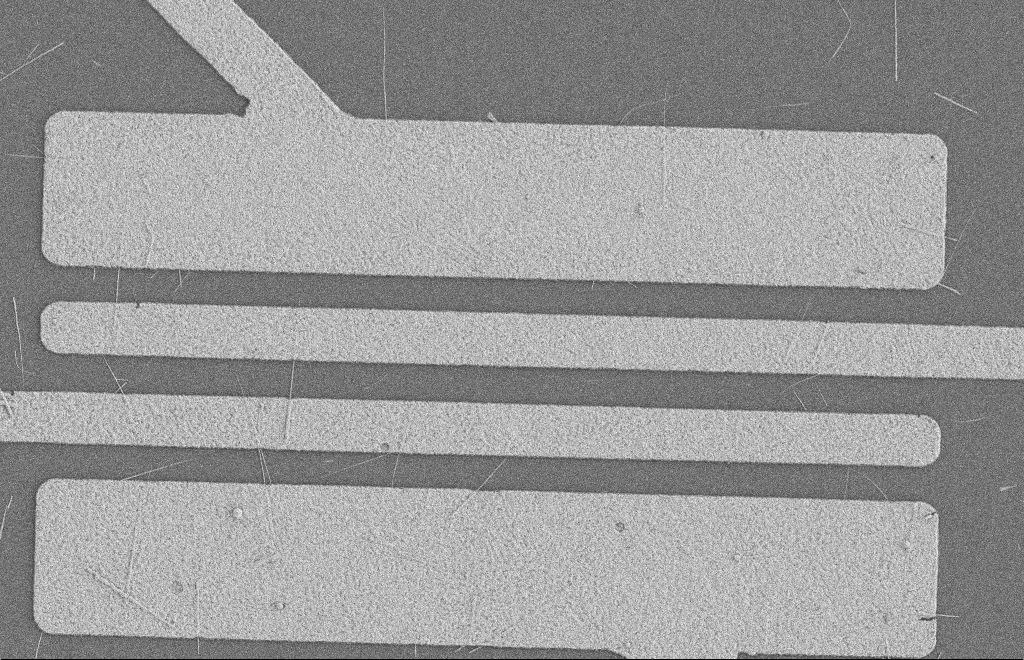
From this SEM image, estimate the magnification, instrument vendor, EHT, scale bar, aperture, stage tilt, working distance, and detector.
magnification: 5.42 K X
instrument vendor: Zeiss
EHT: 2 kV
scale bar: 2000 nm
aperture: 20 µm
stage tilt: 0°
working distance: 8 mm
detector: SE2